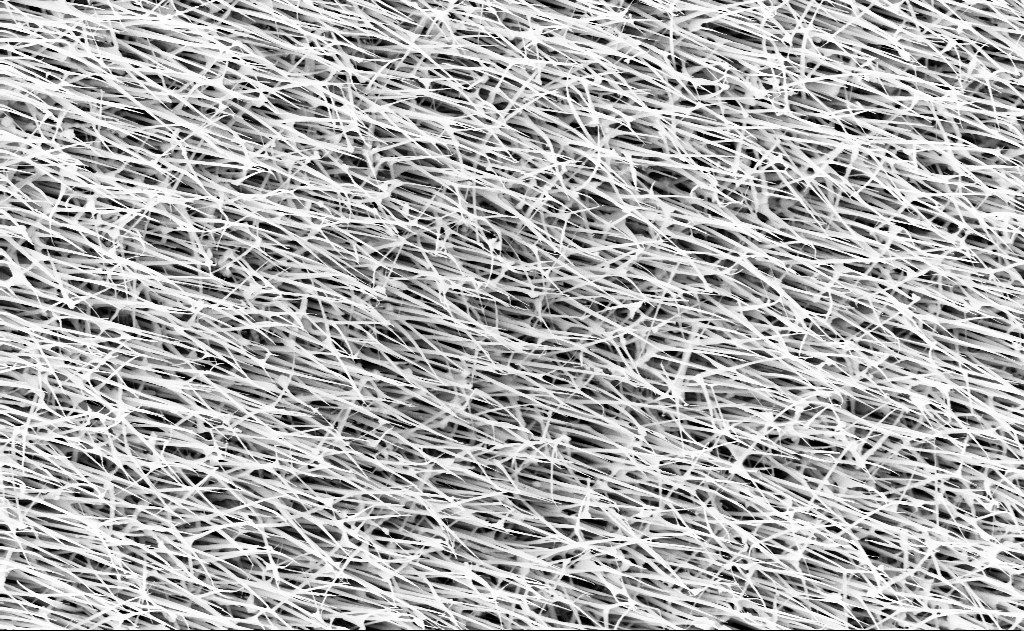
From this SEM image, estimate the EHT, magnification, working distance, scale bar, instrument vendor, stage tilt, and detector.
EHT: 10 kV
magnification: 20 K X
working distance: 13 mm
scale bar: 2000 nm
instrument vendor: Zeiss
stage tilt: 0°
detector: InLens